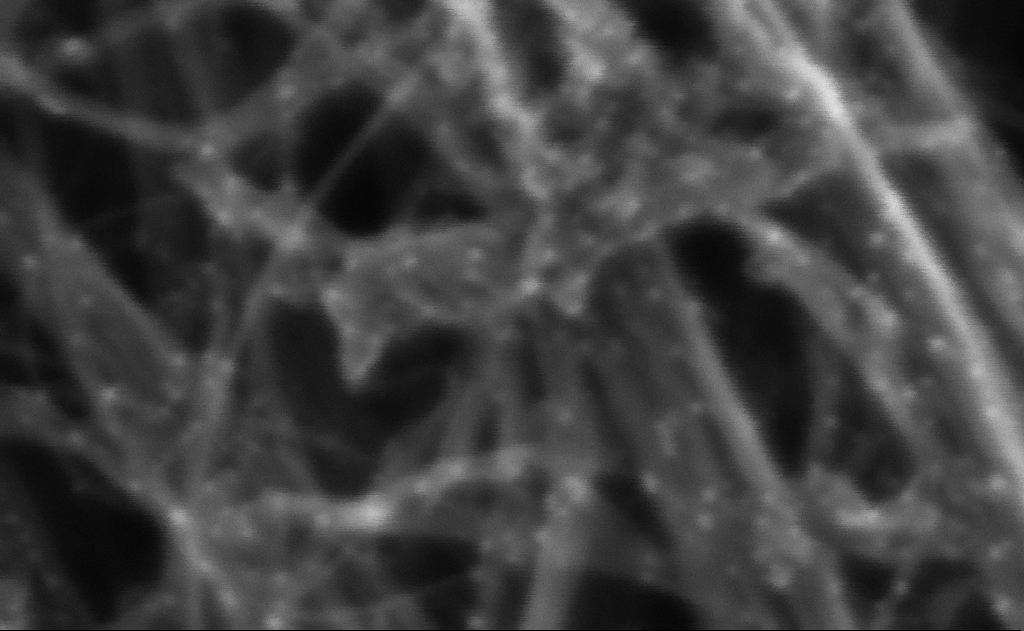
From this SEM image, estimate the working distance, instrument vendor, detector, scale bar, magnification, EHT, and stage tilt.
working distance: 3 mm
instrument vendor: Zeiss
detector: InLens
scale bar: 20 nm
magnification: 1612.06 K X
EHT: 10 kV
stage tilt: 0°